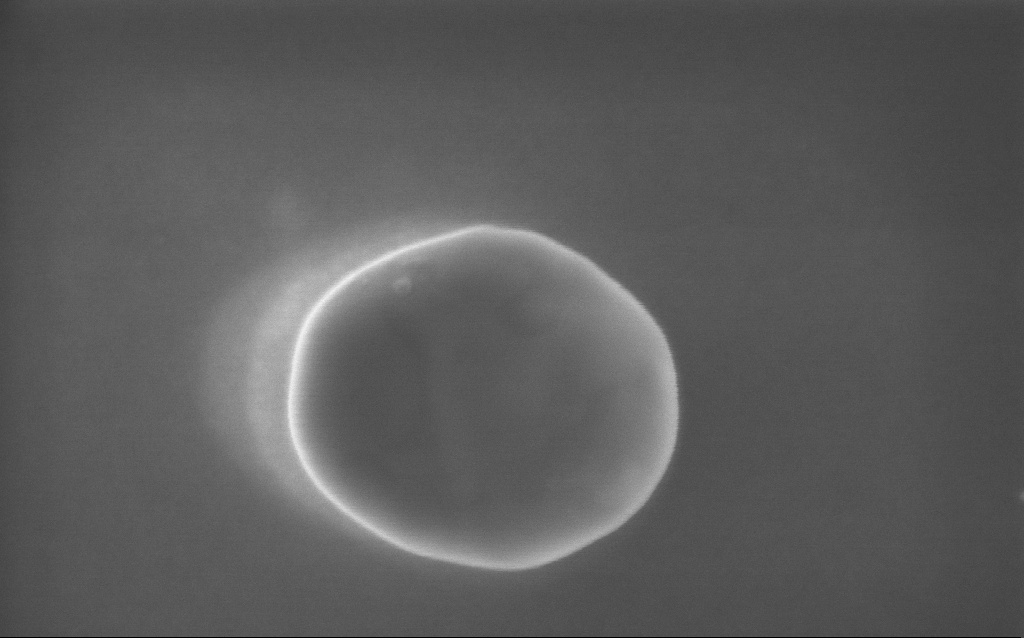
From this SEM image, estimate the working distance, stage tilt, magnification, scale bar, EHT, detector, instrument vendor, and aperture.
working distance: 3 mm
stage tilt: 0°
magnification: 151 K X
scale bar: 200 nm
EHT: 5 kV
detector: InLens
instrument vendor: Zeiss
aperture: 30 µm